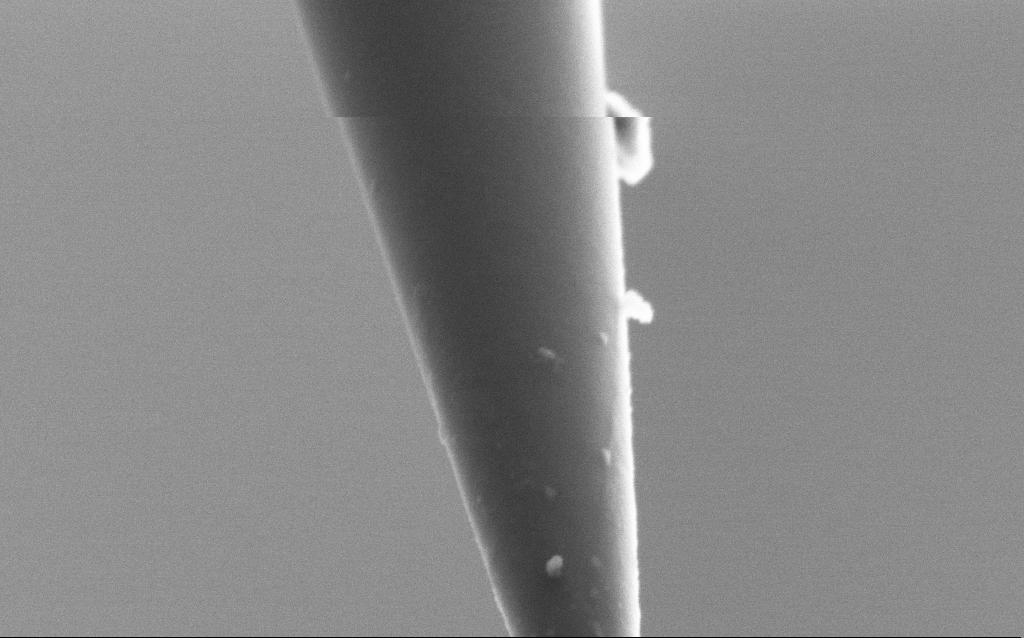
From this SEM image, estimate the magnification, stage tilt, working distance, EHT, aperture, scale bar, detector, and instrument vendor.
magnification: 100 K X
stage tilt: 45°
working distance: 6 mm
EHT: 2.5 kV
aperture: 30 µm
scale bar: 200 nm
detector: SE2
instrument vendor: Zeiss